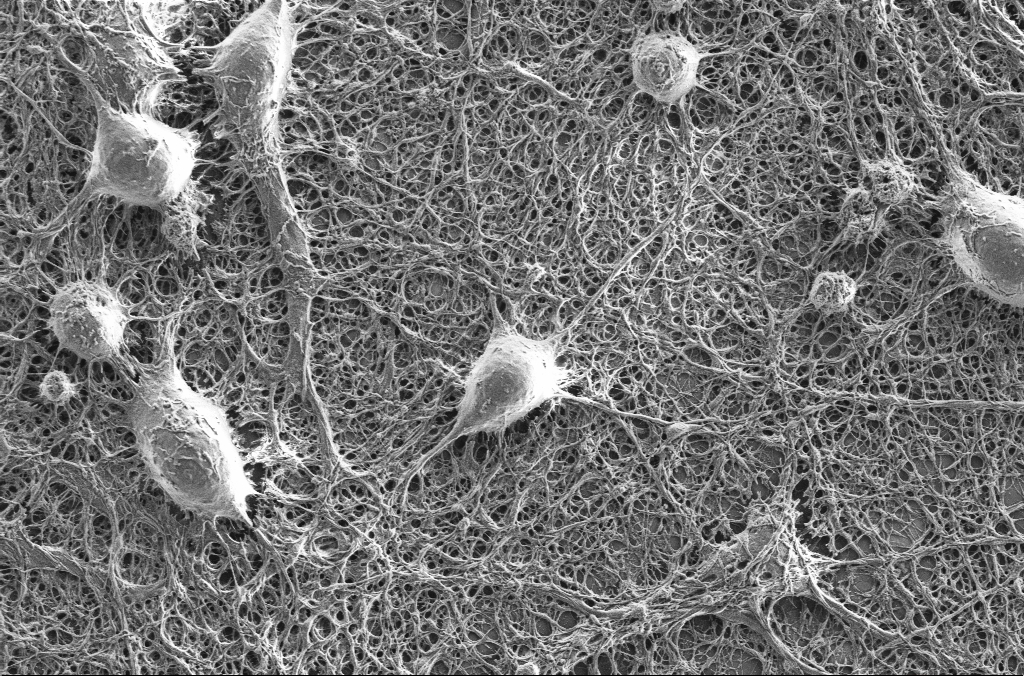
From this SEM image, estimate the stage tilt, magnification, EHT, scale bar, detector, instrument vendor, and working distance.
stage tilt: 0°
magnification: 5 K X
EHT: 2 kV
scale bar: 10000 nm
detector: SE2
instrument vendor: Zeiss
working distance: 4.1 mm